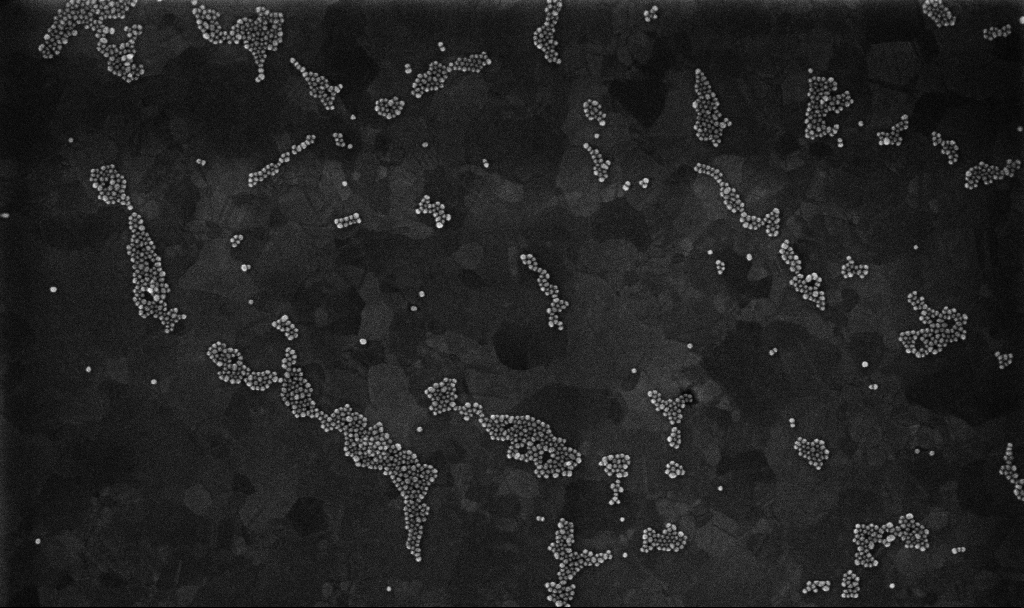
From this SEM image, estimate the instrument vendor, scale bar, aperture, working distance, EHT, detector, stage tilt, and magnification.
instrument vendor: Zeiss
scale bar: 200 nm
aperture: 30 µm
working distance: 3.4 mm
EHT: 10 kV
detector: InLens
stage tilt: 0°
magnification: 100 K X